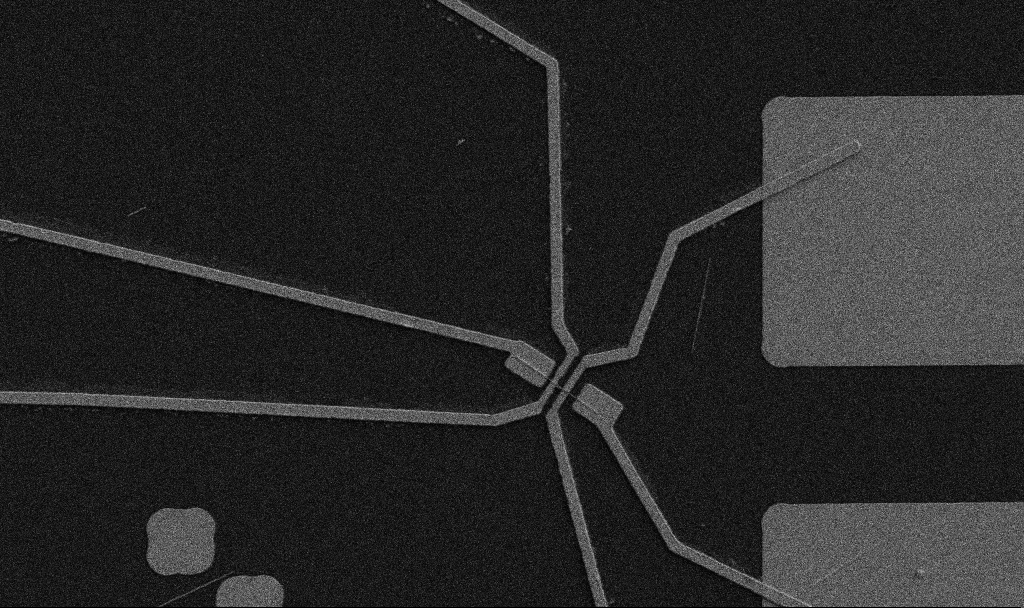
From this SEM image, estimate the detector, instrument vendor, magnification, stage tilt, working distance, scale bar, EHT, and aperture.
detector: SE2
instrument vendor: Zeiss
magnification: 5 K X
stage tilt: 0°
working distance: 10.7 mm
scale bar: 10000 nm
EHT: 5 kV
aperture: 30 µm